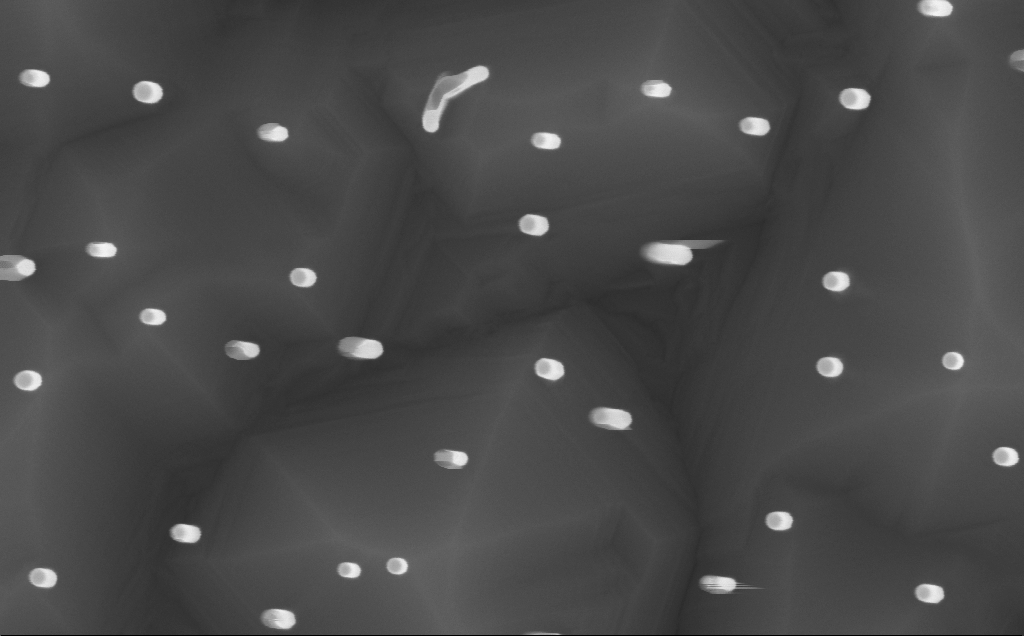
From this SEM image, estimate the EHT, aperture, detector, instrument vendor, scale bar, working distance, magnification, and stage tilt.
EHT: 10 kV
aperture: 30 µm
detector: InLens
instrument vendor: Zeiss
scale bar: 100 nm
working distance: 7 mm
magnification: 135.95 K X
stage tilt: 0.2°